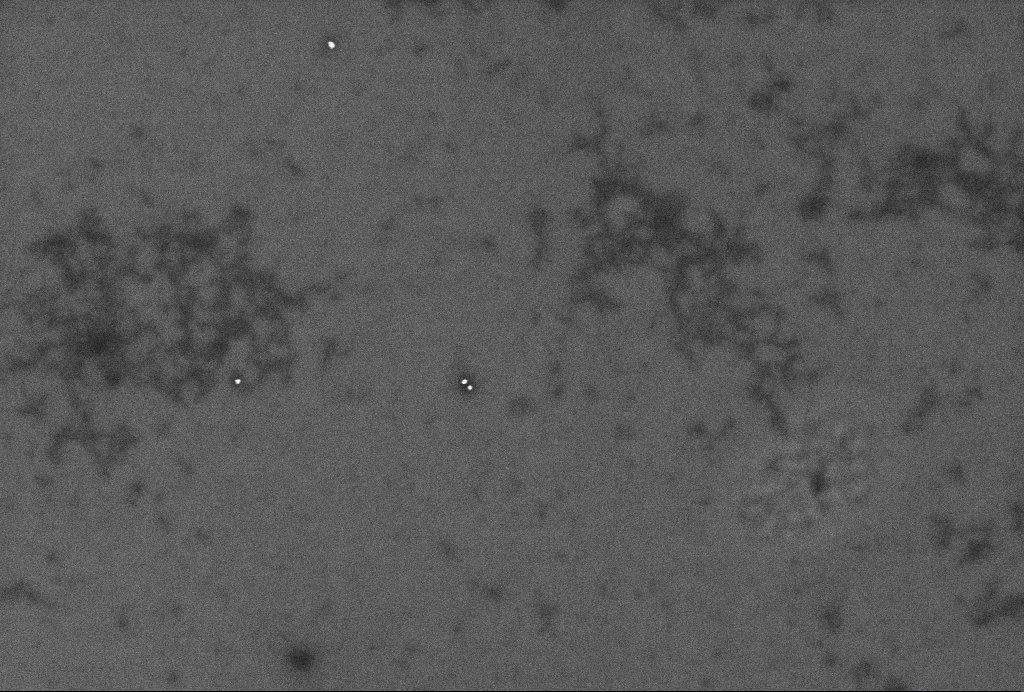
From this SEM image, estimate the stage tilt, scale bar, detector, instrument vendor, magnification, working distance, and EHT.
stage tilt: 0°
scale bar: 200 nm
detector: InLens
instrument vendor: Zeiss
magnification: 62.65 K X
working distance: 3.3 mm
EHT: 2 kV